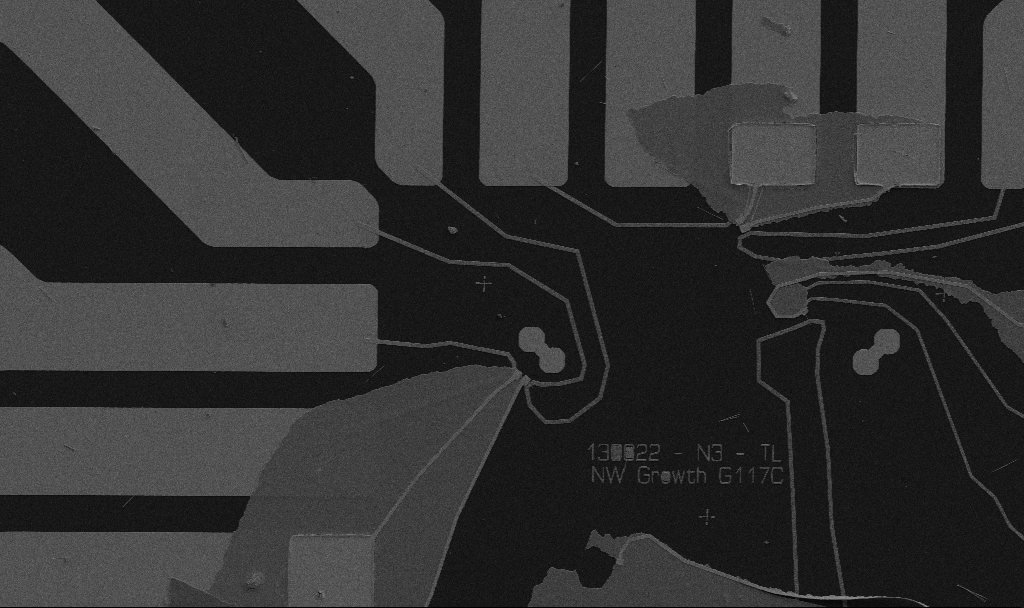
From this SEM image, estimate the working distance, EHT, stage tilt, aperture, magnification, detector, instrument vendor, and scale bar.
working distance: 10.7 mm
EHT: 5 kV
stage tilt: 0°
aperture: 30 µm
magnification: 1.53 K X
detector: SE2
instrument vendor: Zeiss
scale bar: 20000 nm